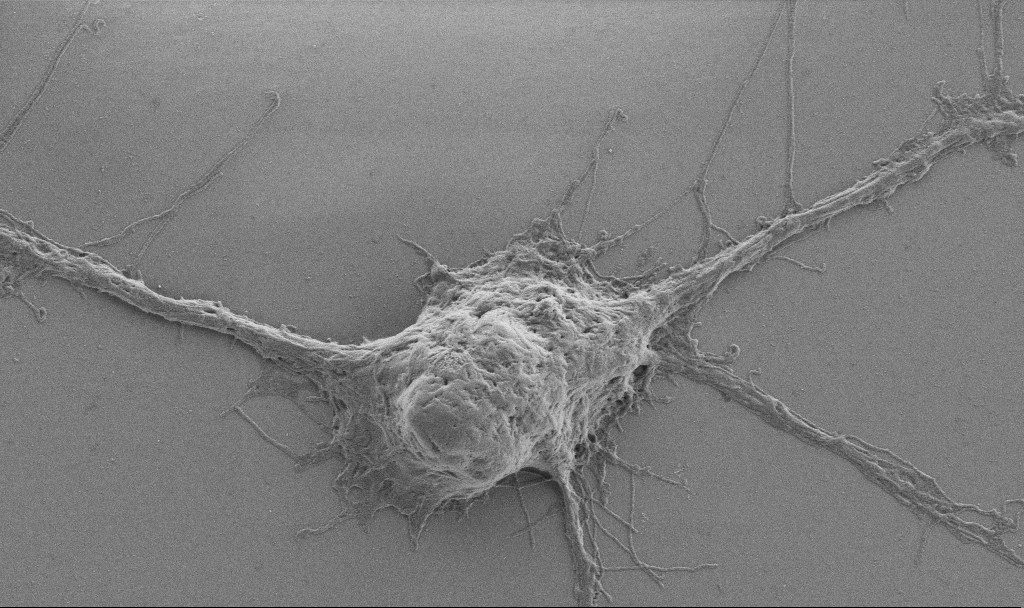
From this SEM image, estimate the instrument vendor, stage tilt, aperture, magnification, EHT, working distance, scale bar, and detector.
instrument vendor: Zeiss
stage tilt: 0°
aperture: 30 µm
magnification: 10 K X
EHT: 0.9 kV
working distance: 6.9 mm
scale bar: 2000 nm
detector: SE2